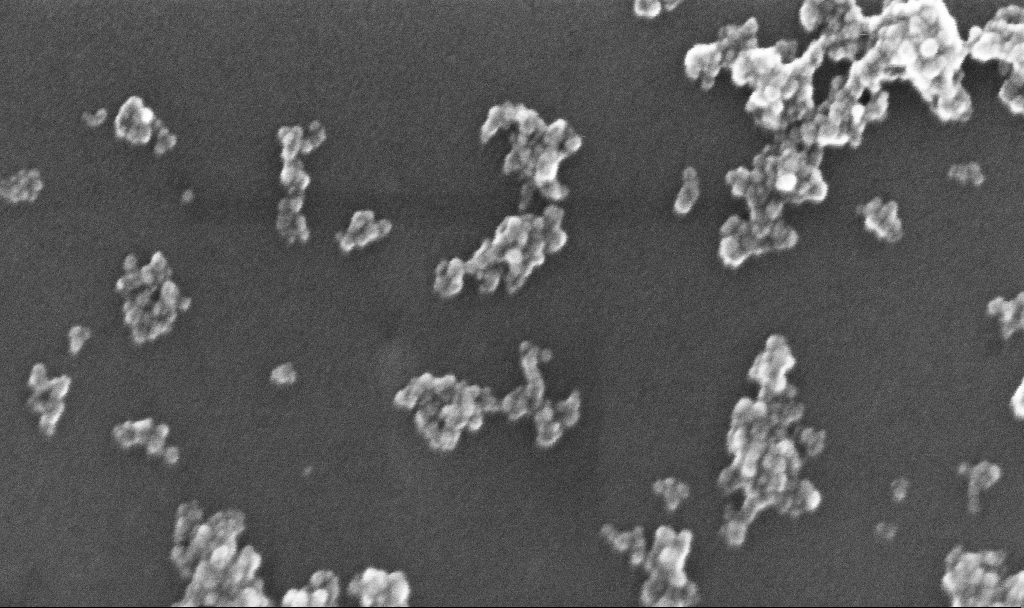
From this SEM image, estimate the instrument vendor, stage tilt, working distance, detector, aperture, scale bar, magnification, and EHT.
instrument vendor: Zeiss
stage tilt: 0°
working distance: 5.2 mm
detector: InLens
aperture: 30 µm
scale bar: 100 nm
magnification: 266.16 K X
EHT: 10 kV